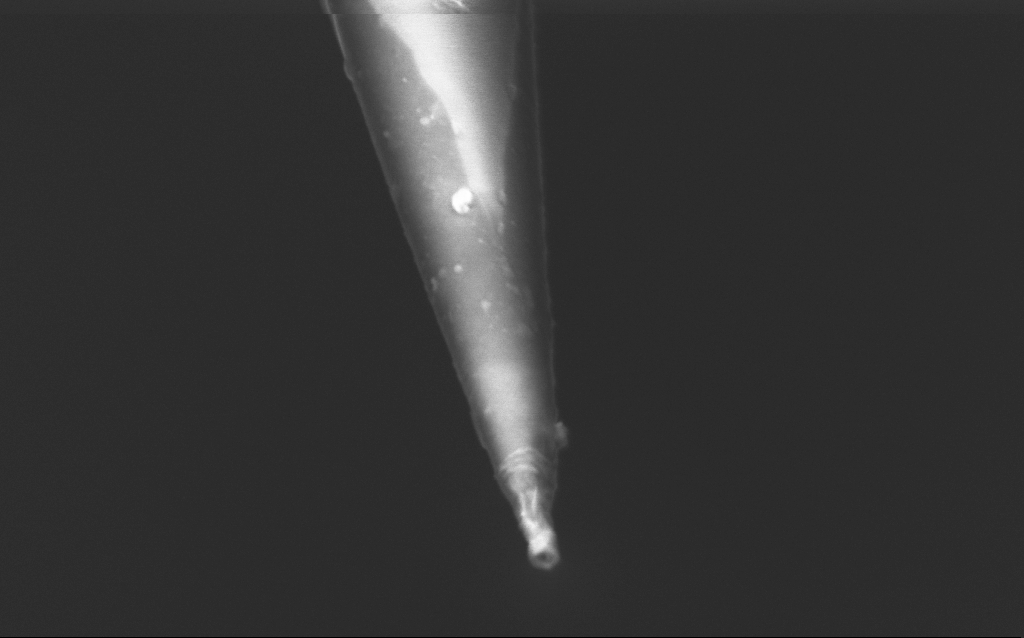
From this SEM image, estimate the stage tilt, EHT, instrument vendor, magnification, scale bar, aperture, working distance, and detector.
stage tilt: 45°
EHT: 1.5 kV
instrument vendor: Zeiss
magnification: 100 K X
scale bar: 200 nm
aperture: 30 µm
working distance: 6 mm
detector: InLens